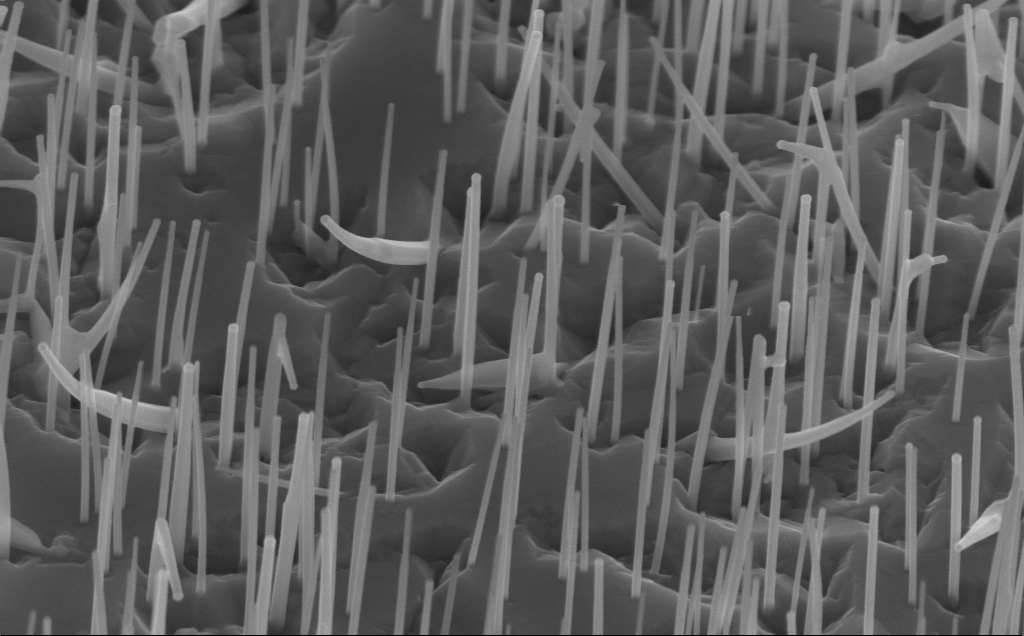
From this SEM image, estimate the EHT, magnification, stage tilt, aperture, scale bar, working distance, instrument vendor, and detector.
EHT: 10 kV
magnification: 80 K X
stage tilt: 45°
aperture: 30 µm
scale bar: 200 nm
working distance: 5 mm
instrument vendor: Zeiss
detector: InLens